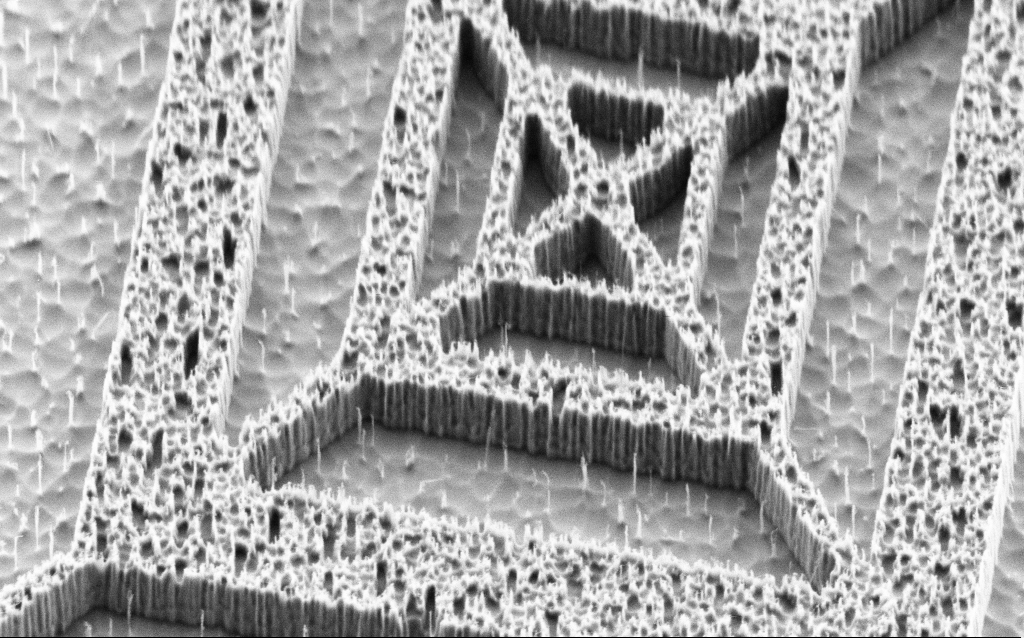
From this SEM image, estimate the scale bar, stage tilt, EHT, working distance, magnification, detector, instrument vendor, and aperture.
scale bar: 1000 nm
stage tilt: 45°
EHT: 2 kV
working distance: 7 mm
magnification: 30.29 K X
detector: SE2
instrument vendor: Zeiss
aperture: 30 µm